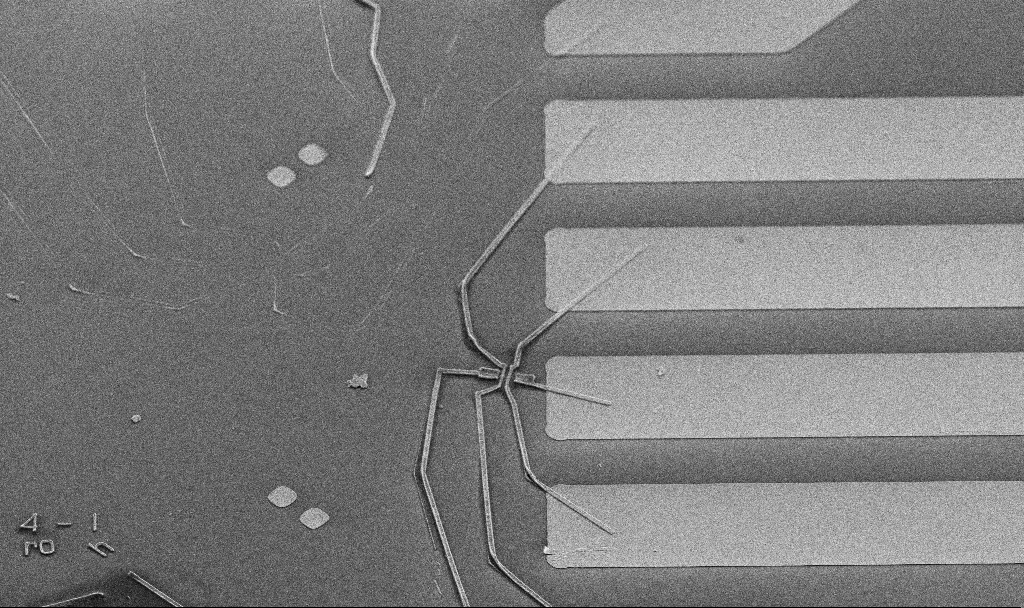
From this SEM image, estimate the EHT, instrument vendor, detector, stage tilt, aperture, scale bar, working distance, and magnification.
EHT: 5 kV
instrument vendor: Zeiss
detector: SE2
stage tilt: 45°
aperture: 30 µm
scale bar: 20000 nm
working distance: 12.4 mm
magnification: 2.25 K X